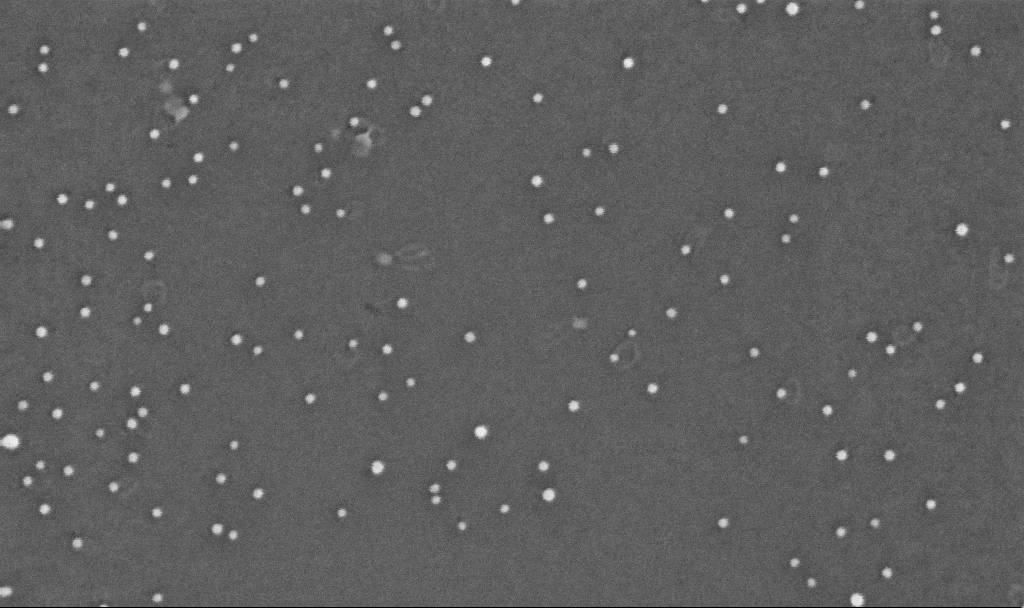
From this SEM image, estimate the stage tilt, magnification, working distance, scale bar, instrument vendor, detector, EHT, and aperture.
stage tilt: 0°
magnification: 350 K X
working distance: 3.3 mm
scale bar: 100 nm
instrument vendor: Zeiss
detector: InLens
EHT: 10 kV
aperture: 30 µm